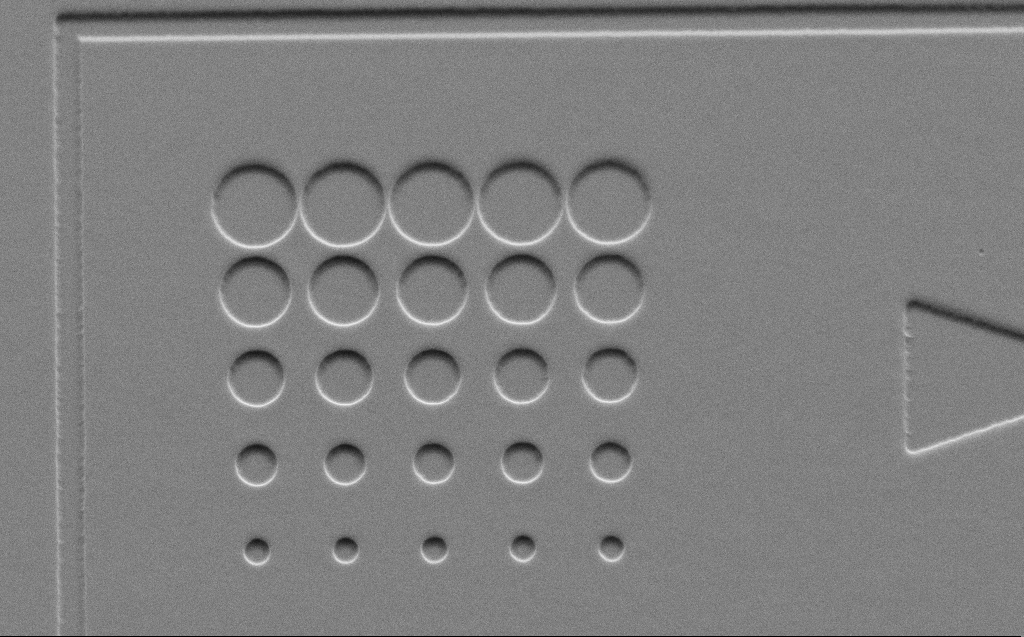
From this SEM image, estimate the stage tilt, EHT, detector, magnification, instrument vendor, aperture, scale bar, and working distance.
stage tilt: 45°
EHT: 3 kV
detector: SE2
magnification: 5.46 K X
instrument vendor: Zeiss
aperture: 30 µm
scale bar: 10000 nm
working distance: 5 mm